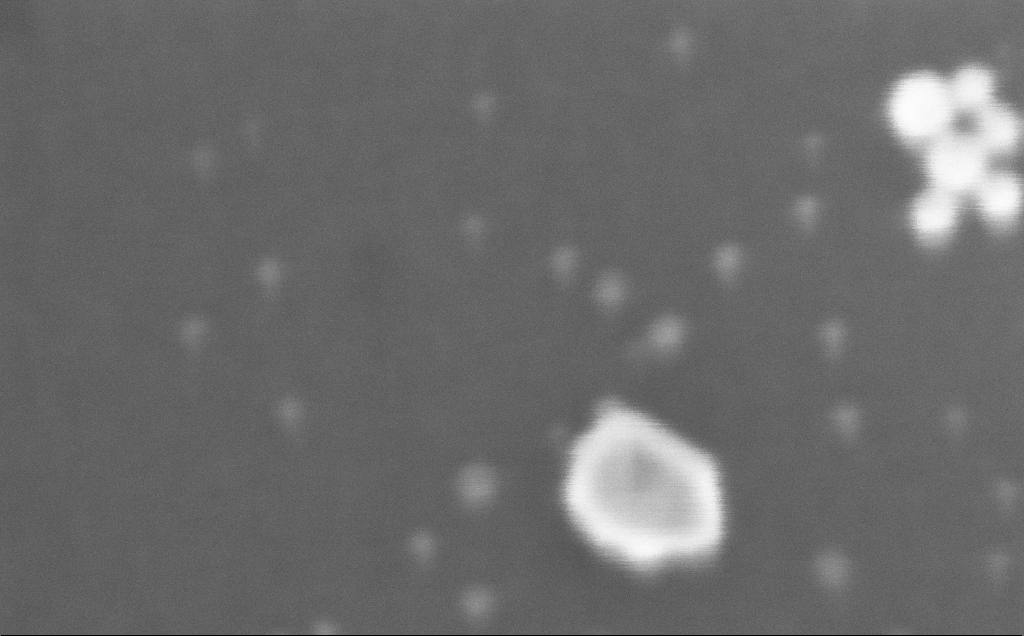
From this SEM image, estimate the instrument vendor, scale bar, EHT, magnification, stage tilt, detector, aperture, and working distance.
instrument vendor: Zeiss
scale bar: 20 nm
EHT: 20 kV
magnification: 1717.56 K X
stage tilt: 0°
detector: InLens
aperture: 30 µm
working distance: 3 mm